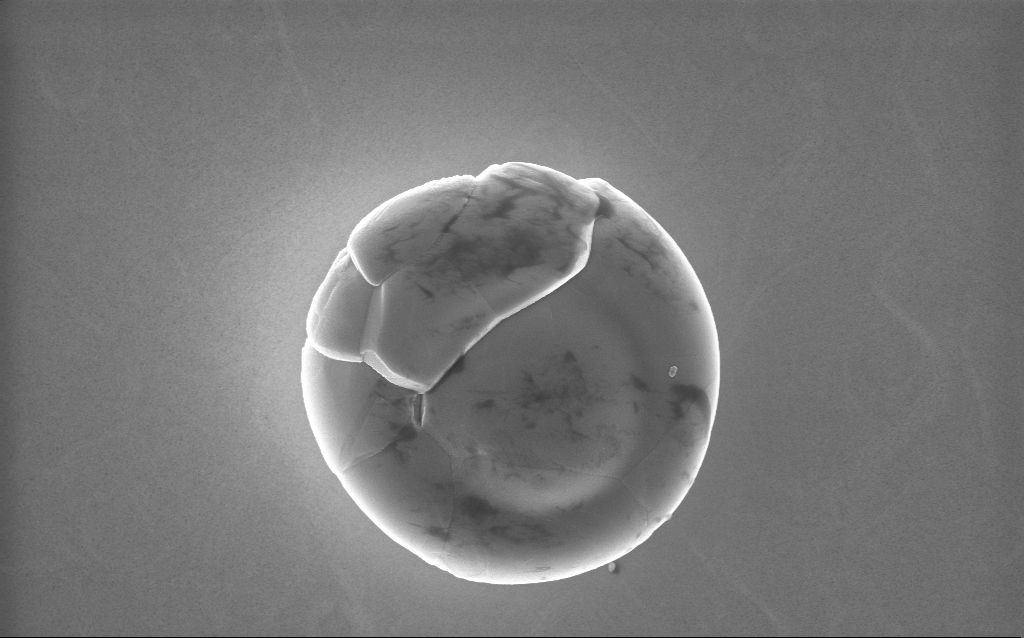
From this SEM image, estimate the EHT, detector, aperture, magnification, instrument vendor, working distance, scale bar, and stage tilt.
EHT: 10 kV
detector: InLens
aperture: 30 µm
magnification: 37.12 K X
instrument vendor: Zeiss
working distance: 2 mm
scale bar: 1000 nm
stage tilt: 0°